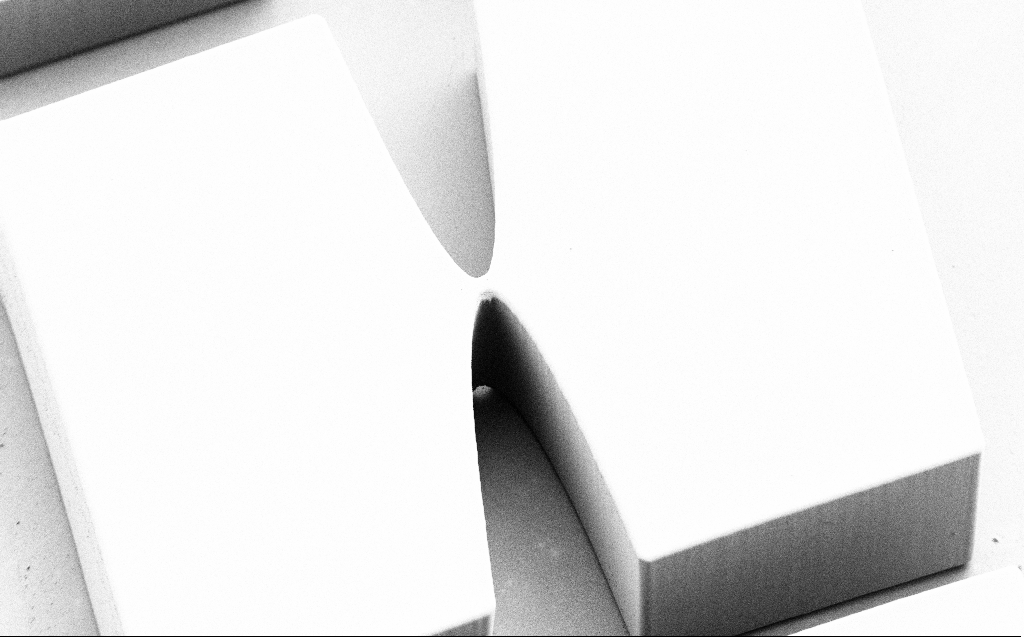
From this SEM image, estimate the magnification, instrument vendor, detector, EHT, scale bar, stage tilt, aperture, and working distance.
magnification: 0.861 K X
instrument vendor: Zeiss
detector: SE2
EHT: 1 kV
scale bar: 20000 nm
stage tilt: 45°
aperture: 30 µm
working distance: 6 mm